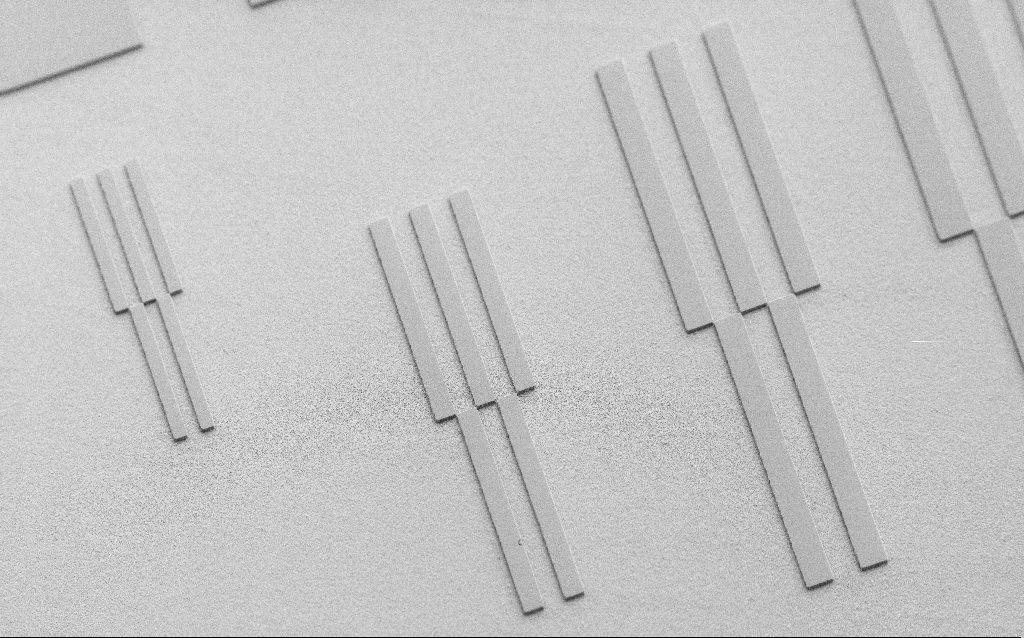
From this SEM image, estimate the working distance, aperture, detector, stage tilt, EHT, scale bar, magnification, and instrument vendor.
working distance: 7 mm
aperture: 30 µm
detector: SE2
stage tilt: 45°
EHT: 3 kV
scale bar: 100000 nm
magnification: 0.262 K X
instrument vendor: Zeiss